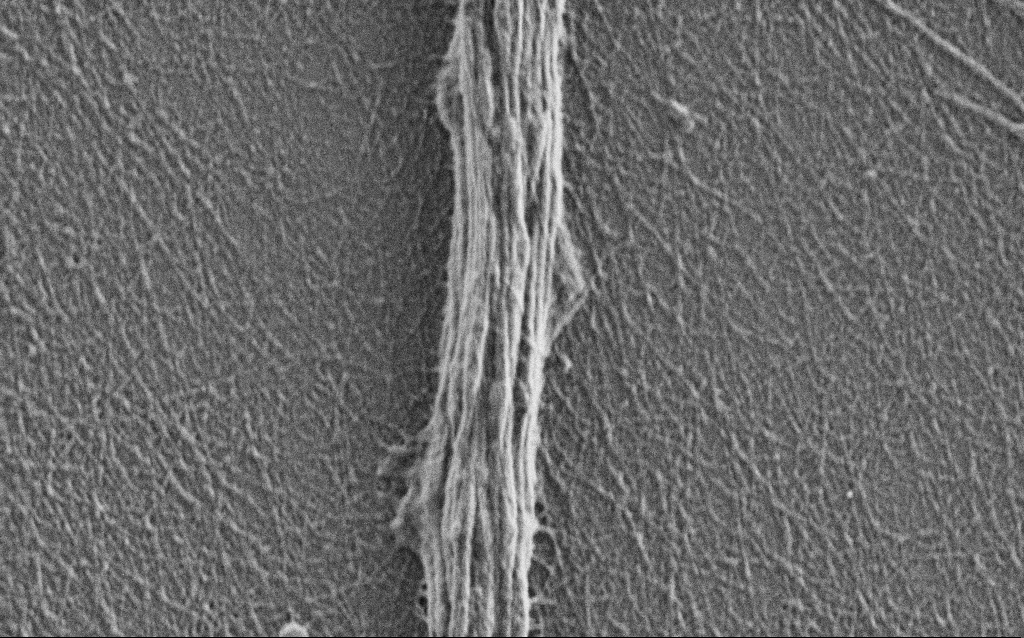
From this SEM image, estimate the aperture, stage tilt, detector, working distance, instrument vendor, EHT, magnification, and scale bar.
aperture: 30 µm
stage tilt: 0°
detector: SE2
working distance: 4 mm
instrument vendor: Zeiss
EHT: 1 kV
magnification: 25 K X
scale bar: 1000 nm